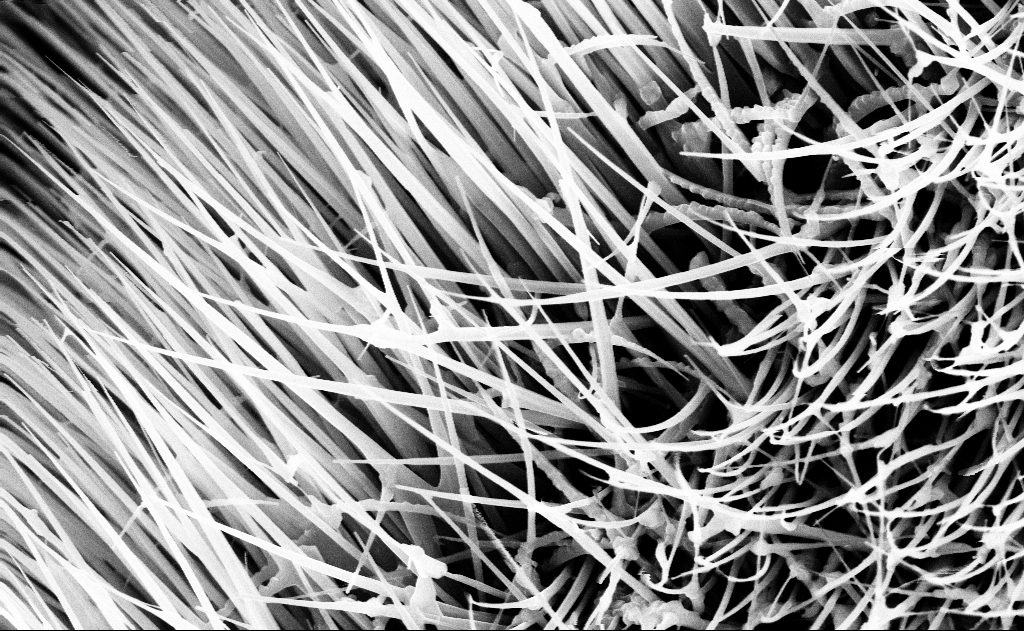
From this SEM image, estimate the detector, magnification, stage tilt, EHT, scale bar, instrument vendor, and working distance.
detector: InLens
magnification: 40 K X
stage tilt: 0°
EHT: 10 kV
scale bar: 1000 nm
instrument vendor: Zeiss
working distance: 12 mm